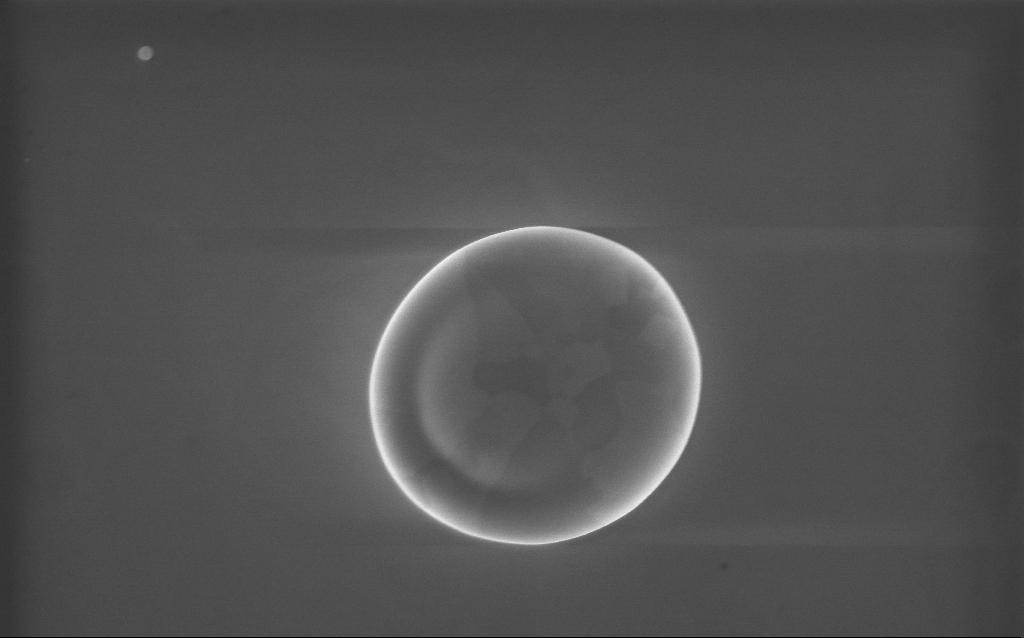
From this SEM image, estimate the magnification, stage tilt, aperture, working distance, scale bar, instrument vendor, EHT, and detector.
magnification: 36 K X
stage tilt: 0°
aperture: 30 µm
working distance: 2 mm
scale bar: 1000 nm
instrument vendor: Zeiss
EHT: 10 kV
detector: InLens